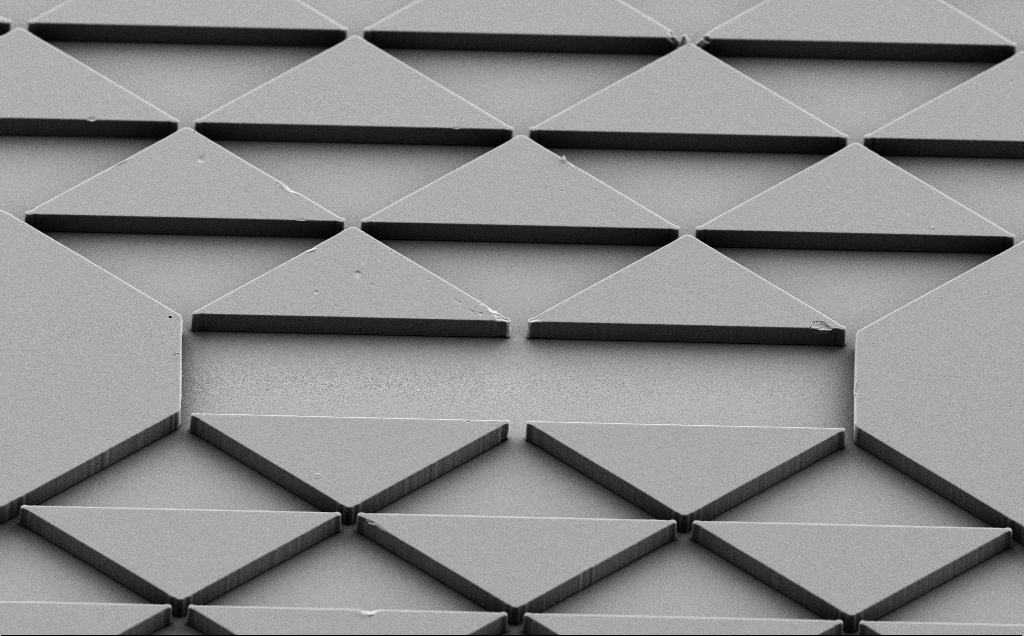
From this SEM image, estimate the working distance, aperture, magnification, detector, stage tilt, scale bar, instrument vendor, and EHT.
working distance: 8 mm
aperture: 30 µm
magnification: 1.32 K X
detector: SE2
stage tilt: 40°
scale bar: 10000 nm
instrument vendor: Zeiss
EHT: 5 kV